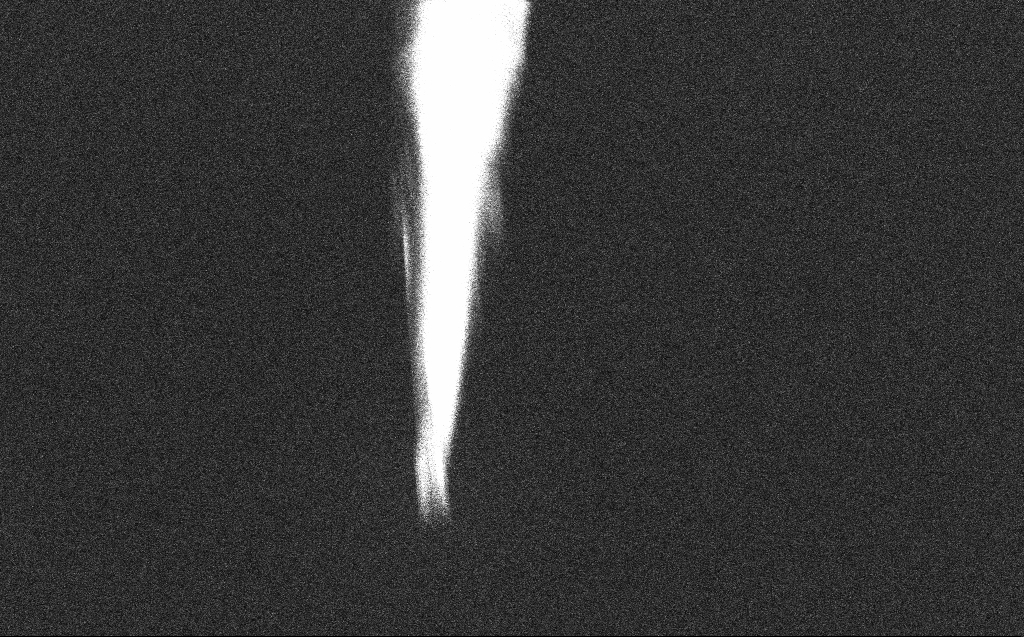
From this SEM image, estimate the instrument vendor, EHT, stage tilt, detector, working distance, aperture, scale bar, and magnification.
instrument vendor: Zeiss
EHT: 2 kV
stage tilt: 45°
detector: InLens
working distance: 4 mm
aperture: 20 µm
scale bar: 2000 nm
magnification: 22.85 K X